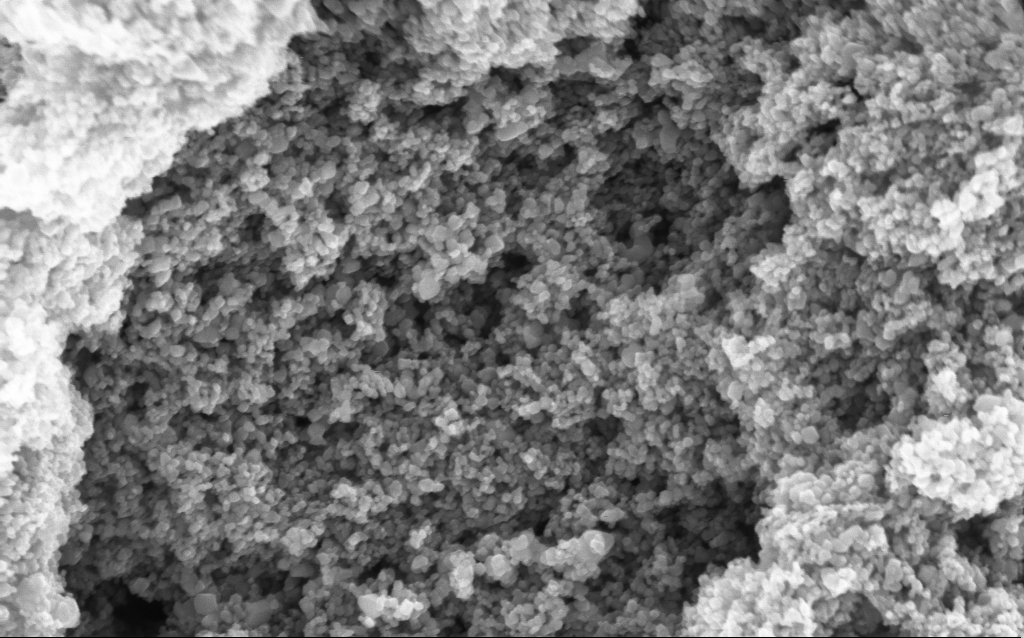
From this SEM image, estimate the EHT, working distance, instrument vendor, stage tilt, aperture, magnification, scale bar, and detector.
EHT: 5 kV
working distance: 4.7 mm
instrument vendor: Zeiss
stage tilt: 0°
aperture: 30 µm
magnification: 114.65 K X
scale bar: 200 nm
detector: InLens